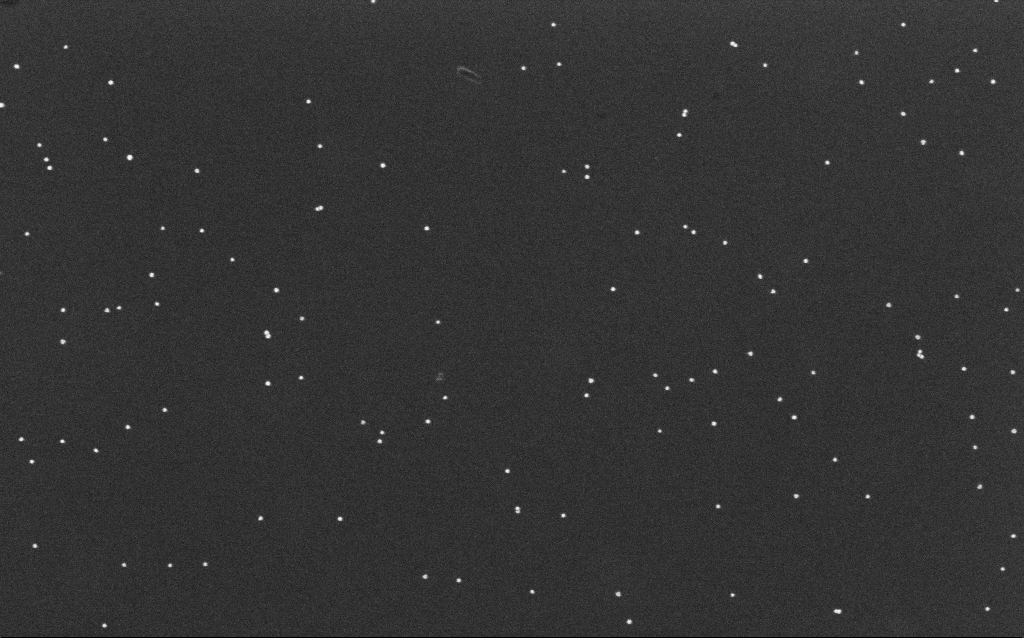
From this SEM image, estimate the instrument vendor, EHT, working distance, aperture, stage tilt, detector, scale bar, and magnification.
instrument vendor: Zeiss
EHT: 10 kV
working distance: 6.4 mm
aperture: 30 µm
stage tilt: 0°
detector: InLens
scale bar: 200 nm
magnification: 100 K X